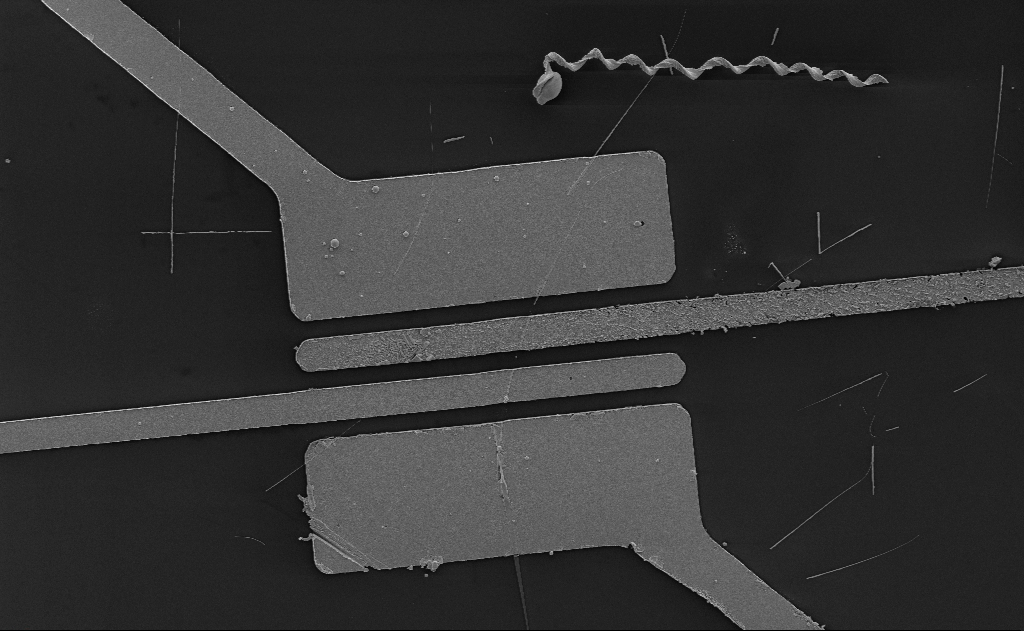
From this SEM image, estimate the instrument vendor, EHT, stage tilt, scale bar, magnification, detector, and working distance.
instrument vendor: Zeiss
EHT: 5 kV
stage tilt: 0°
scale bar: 10000 nm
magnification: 4.67 K X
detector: SE2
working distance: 15 mm